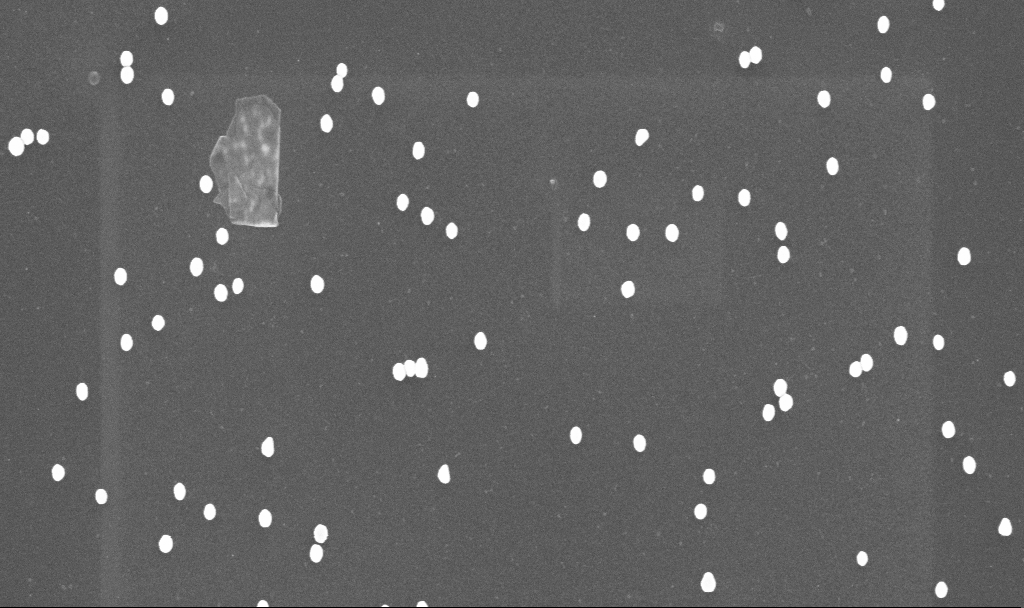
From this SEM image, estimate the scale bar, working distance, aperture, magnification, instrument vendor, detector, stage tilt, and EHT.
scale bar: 1000 nm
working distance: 3.4 mm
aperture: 30 µm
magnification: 70 K X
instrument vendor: Zeiss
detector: InLens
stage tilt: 0°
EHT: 10 kV